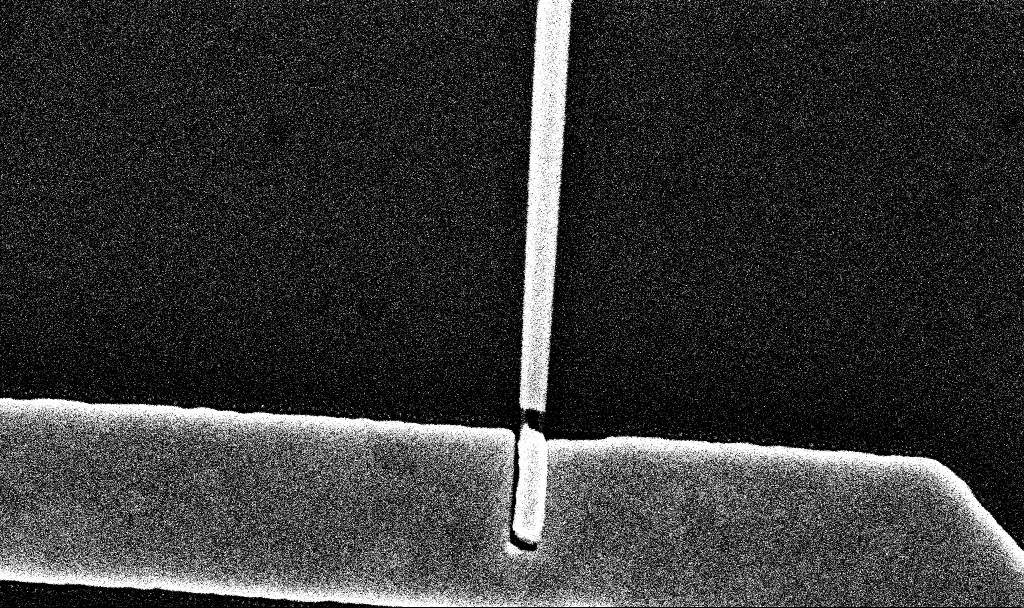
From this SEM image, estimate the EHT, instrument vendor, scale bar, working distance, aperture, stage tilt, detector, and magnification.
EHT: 10 kV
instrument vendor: Zeiss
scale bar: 200 nm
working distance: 7.7 mm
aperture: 30 µm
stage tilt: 0°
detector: InLens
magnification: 72.91 K X